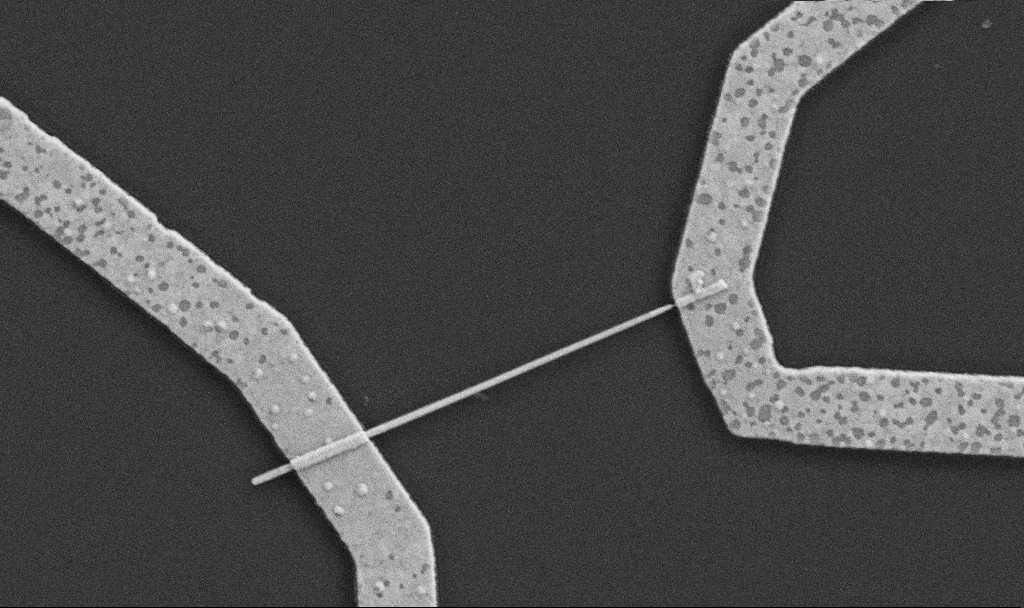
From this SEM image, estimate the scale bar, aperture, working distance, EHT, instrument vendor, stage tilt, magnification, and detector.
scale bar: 1000 nm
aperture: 30 µm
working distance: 8.7 mm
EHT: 5 kV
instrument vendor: Zeiss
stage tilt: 0°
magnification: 30 K X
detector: SE2